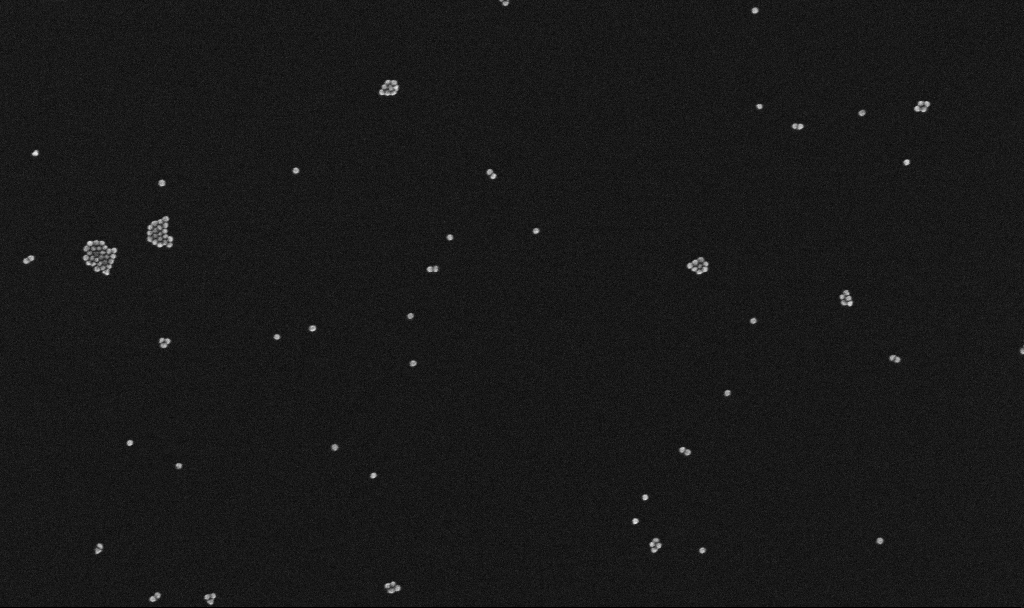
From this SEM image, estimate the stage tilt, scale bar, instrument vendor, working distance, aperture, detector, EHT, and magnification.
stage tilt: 0°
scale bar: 200 nm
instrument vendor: Zeiss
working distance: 3.2 mm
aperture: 30 µm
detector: InLens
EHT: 10 kV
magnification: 100 K X